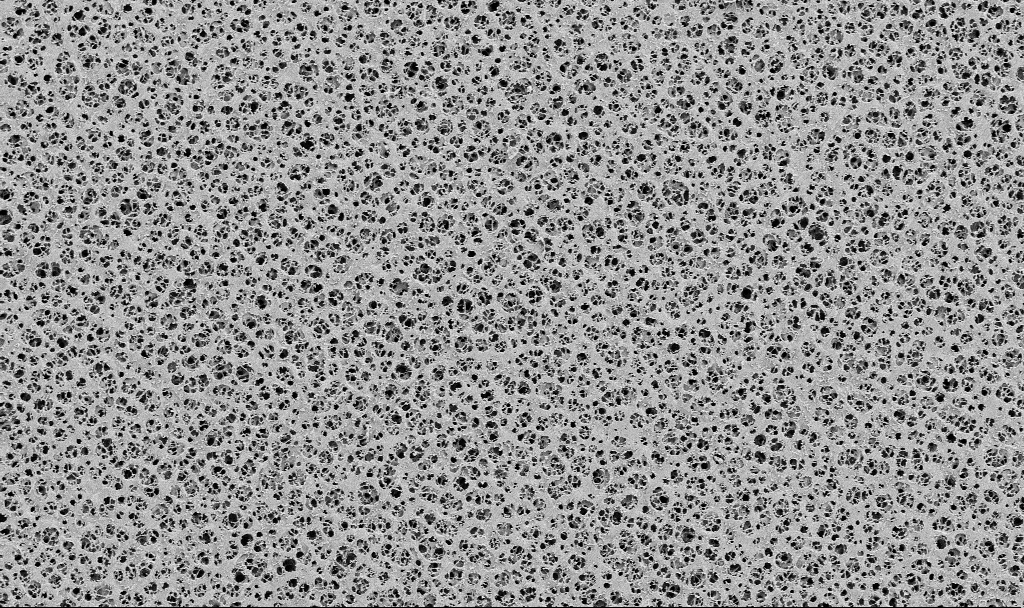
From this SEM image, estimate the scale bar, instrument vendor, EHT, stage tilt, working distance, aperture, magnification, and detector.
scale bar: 10000 nm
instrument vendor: Zeiss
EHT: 2 kV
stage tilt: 0°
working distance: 3.7 mm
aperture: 30 µm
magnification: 2 K X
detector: SE2